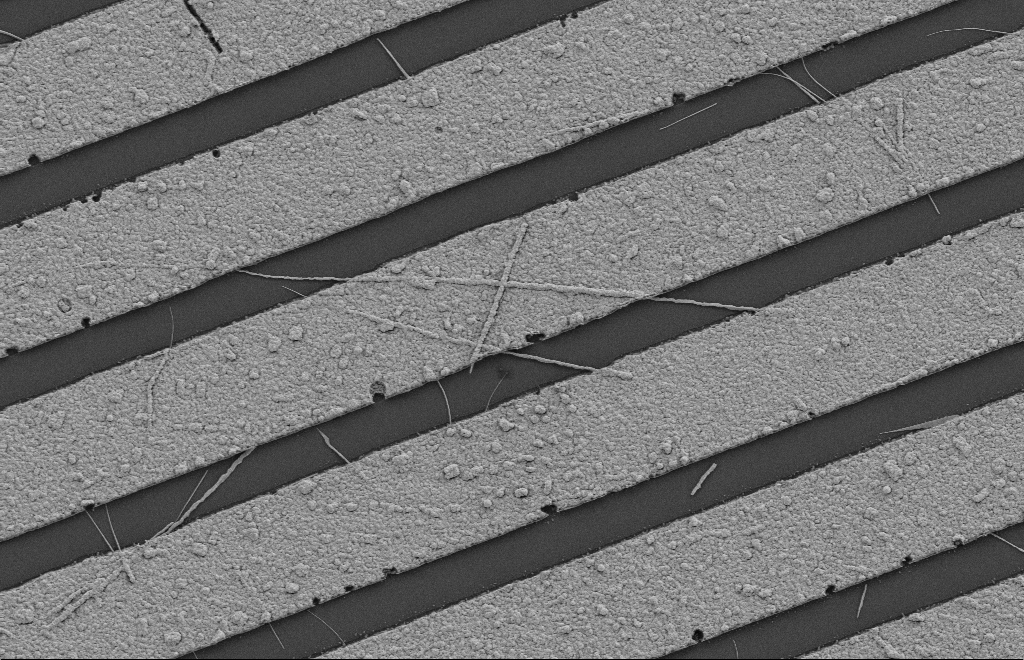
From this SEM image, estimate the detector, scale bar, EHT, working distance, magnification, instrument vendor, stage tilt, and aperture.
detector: SE2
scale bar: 2000 nm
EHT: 2 kV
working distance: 8 mm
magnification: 10.44 K X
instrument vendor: Zeiss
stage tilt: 0°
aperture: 20 µm